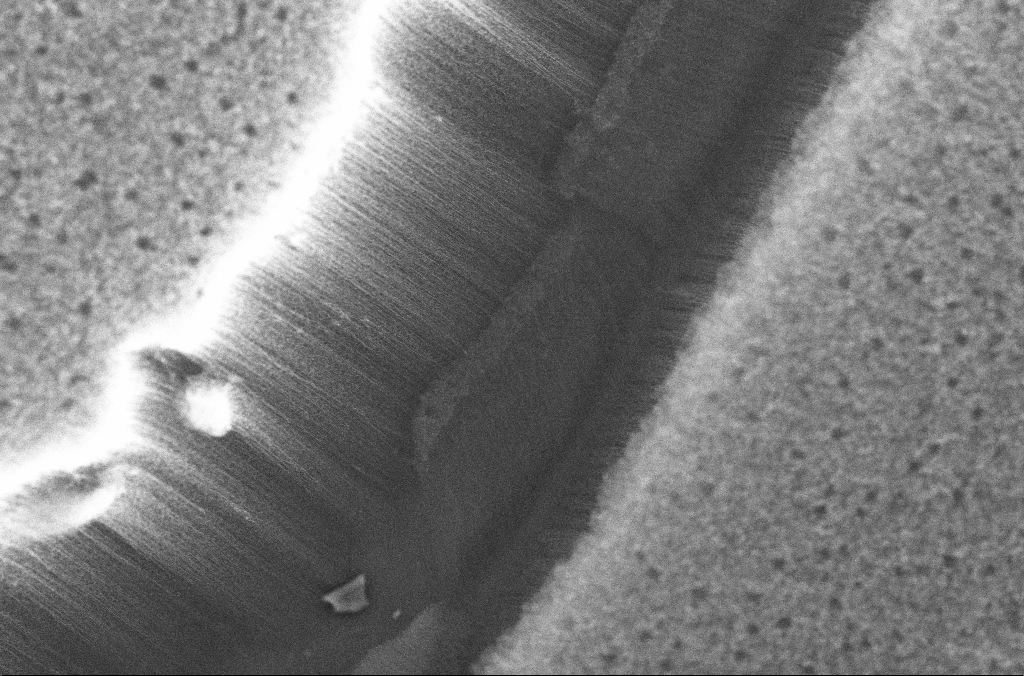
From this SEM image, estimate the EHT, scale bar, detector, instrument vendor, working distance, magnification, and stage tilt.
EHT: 10 kV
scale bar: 10000 nm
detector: InLens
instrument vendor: Zeiss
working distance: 4 mm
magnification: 5 K X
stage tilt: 0°